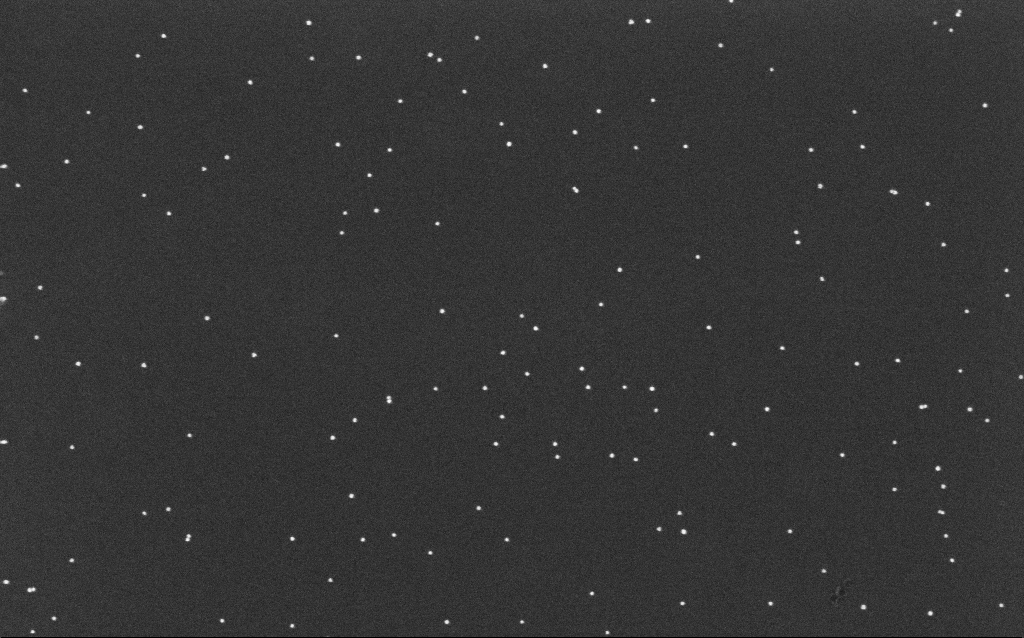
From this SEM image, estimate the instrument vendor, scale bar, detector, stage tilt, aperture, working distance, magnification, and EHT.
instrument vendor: Zeiss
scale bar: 200 nm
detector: InLens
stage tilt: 0°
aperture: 30 µm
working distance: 6.4 mm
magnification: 100 K X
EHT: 10 kV